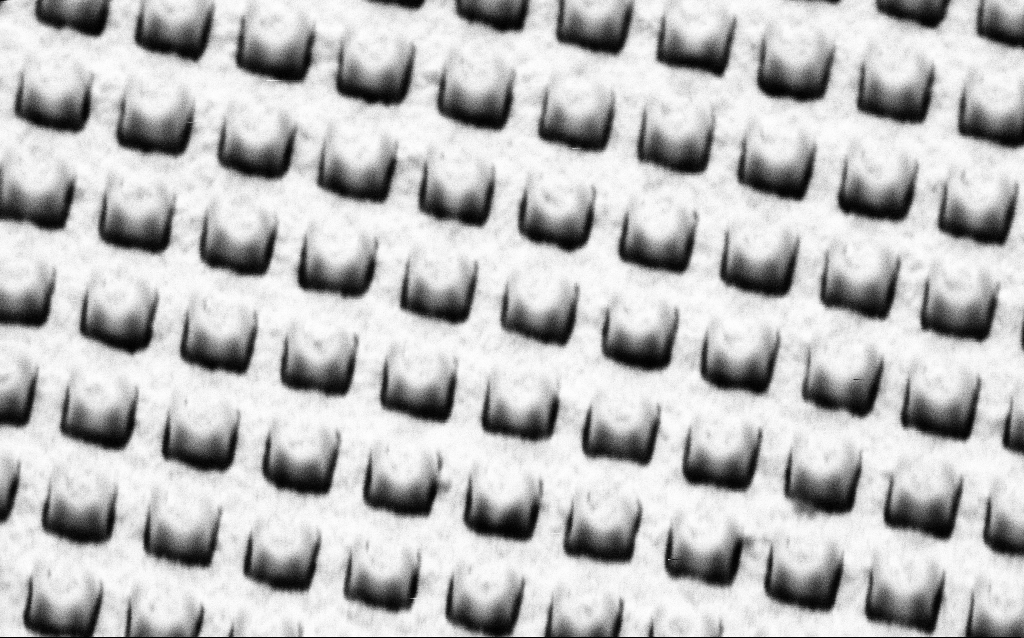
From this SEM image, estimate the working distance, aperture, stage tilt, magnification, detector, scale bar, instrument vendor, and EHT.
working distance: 6.4 mm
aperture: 30 µm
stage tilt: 45°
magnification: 81.21 K X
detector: SE2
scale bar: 200 nm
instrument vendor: Zeiss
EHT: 1.5 kV